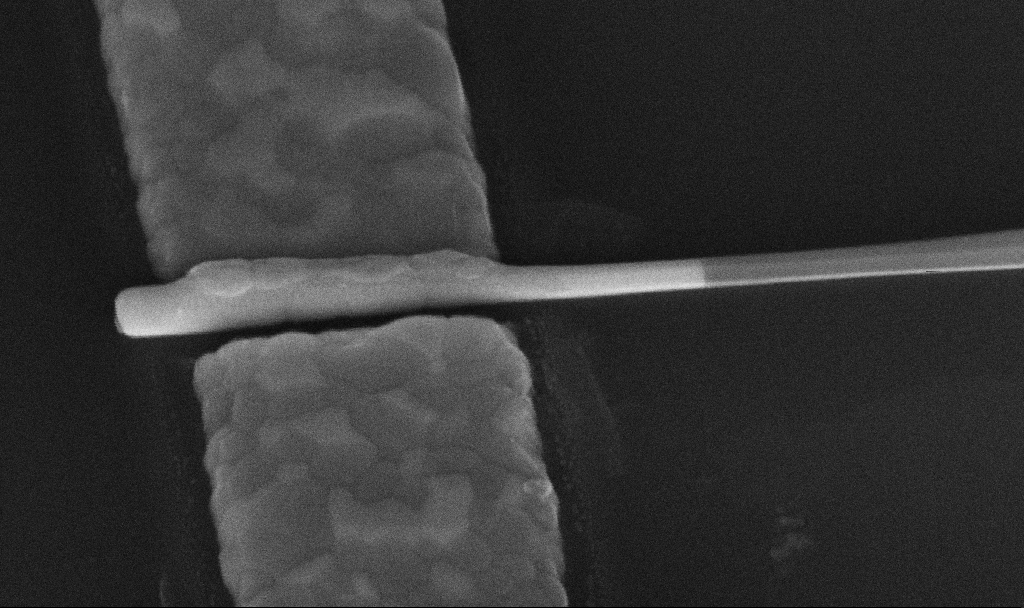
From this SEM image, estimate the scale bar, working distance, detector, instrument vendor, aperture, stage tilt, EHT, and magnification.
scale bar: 200 nm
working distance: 10.6 mm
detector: InLens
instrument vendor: Zeiss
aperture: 30 µm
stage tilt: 45°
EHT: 10 kV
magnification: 173.9 K X